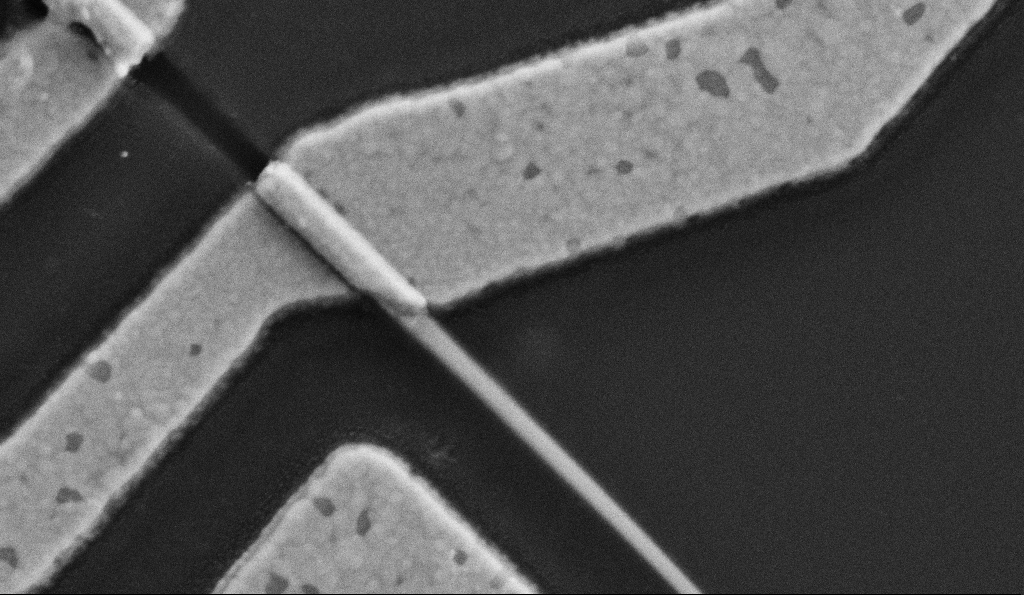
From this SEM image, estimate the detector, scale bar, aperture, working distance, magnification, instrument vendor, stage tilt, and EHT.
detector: SE2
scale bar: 200 nm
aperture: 30 µm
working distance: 7.7 mm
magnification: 100 K X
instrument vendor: Zeiss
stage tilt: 0°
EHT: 5 kV